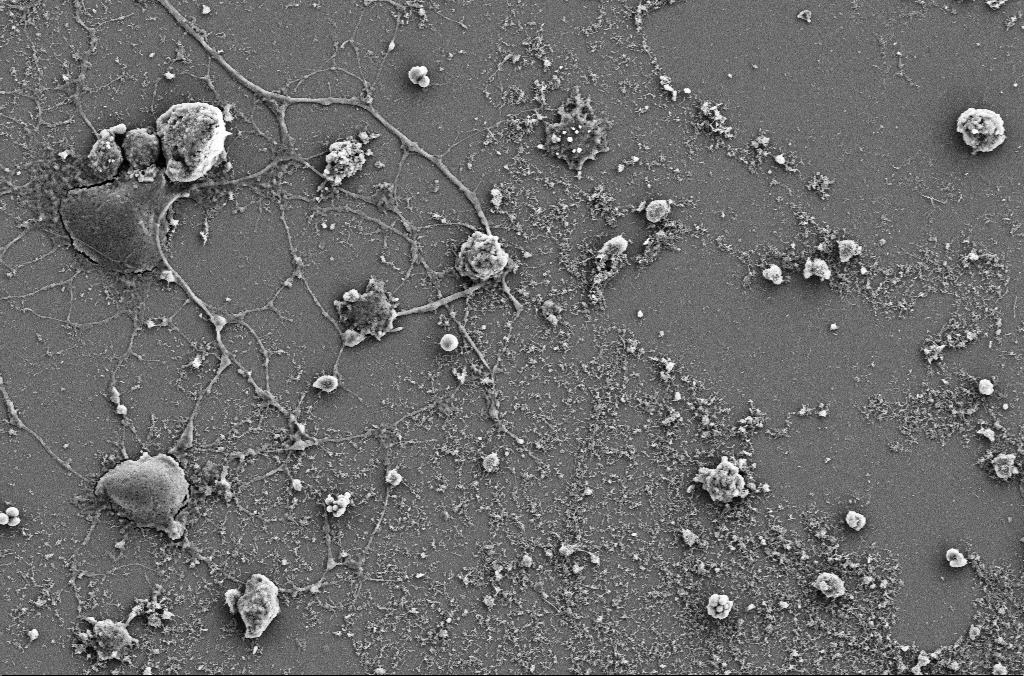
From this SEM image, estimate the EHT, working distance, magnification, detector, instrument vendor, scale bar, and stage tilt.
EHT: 5 kV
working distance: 4 mm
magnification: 4 K X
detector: SE2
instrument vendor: Zeiss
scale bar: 10000 nm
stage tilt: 0°